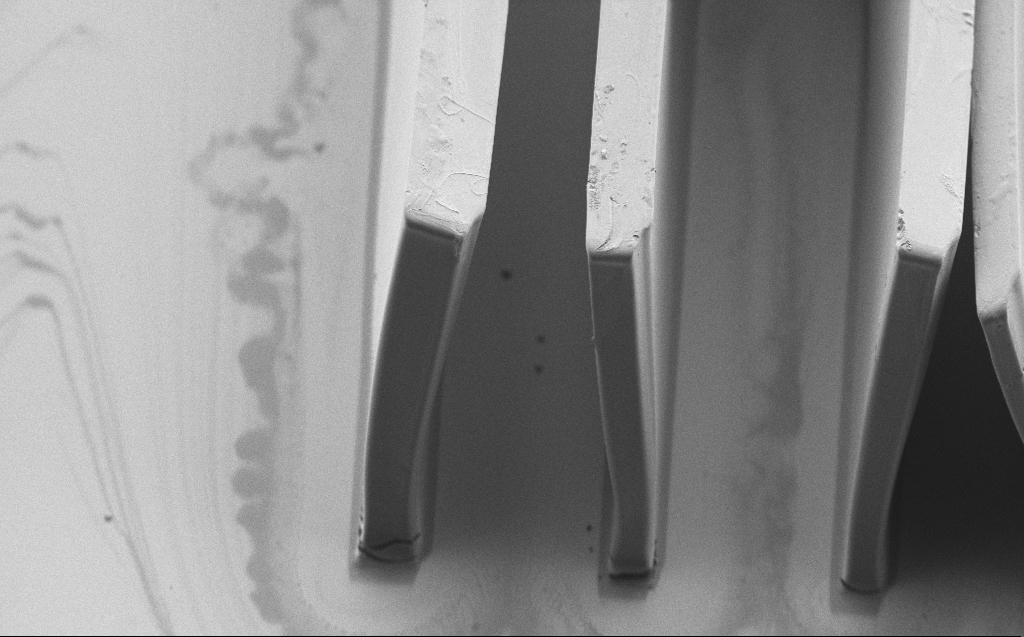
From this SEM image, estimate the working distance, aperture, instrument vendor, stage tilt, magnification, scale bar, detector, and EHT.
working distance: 5 mm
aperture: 30 µm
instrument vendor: Zeiss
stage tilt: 45°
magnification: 1.31 K X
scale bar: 20000 nm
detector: SE2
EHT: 1.2 kV